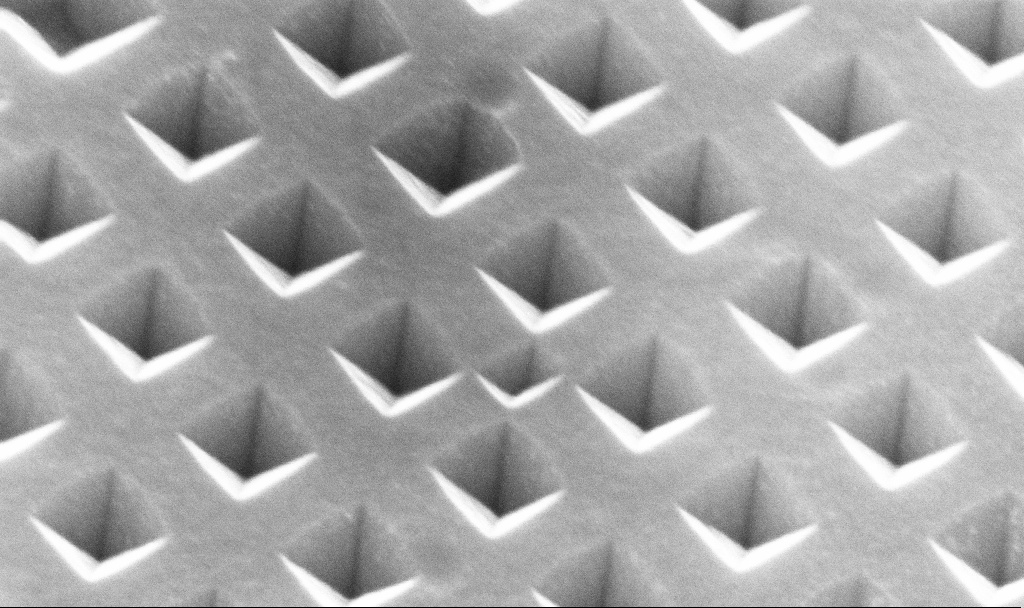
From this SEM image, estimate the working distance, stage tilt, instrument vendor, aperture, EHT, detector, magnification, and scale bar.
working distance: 3.8 mm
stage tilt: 35°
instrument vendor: Zeiss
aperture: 30 µm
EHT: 10 kV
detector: InLens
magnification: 221.14 K X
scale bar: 200 nm